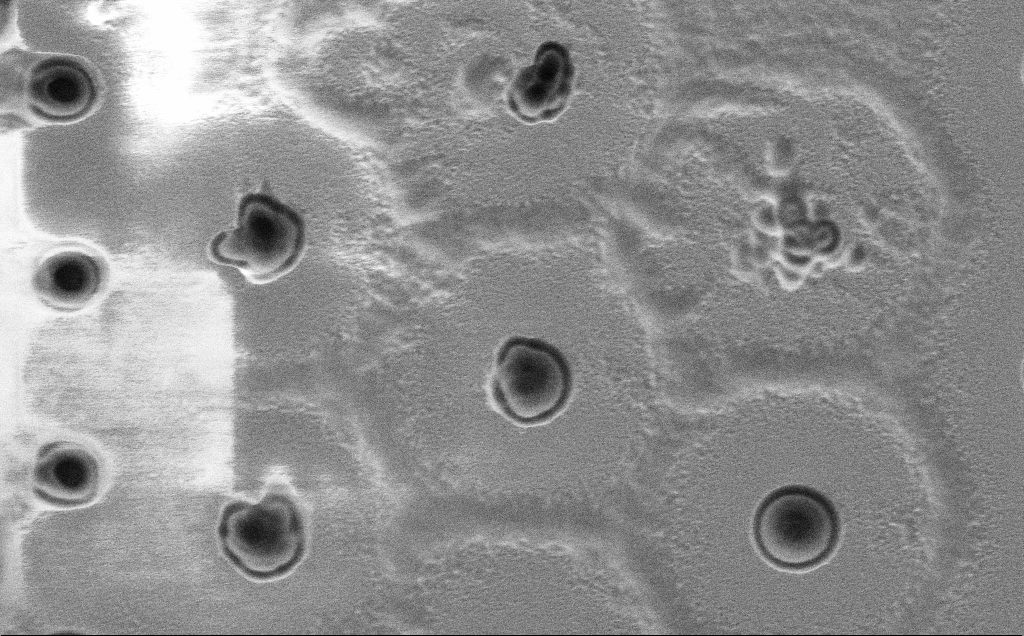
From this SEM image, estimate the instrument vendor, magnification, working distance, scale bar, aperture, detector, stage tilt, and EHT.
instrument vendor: Zeiss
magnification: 11.13 K X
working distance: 7 mm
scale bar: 2000 nm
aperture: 30 µm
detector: SE2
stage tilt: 0°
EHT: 1 kV